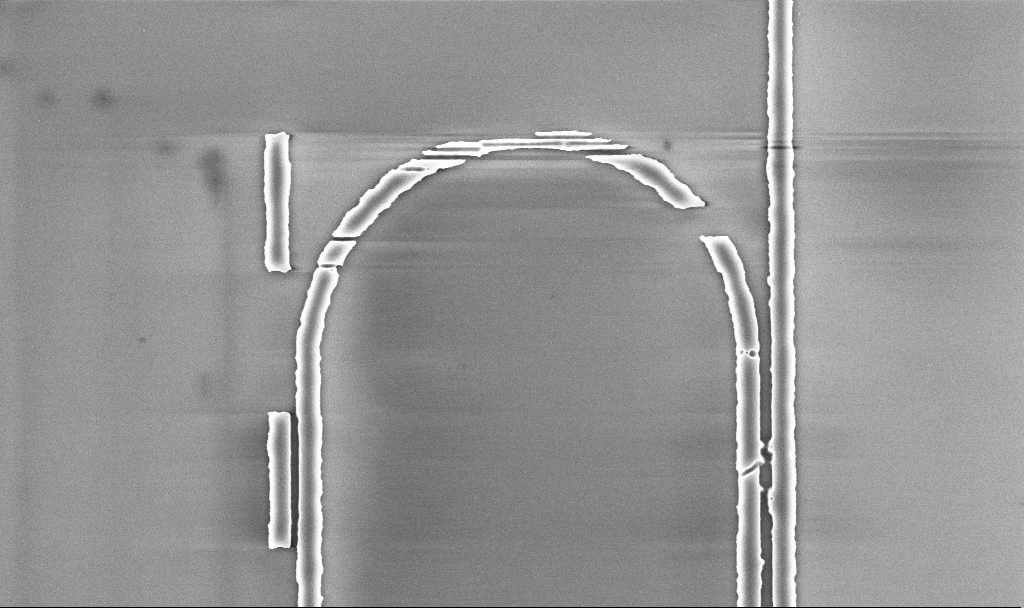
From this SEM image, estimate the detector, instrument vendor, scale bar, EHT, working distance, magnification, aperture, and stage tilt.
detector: InLens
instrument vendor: Zeiss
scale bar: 2000 nm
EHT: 5 kV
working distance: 5.2 mm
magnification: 17.2 K X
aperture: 30 µm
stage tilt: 0°